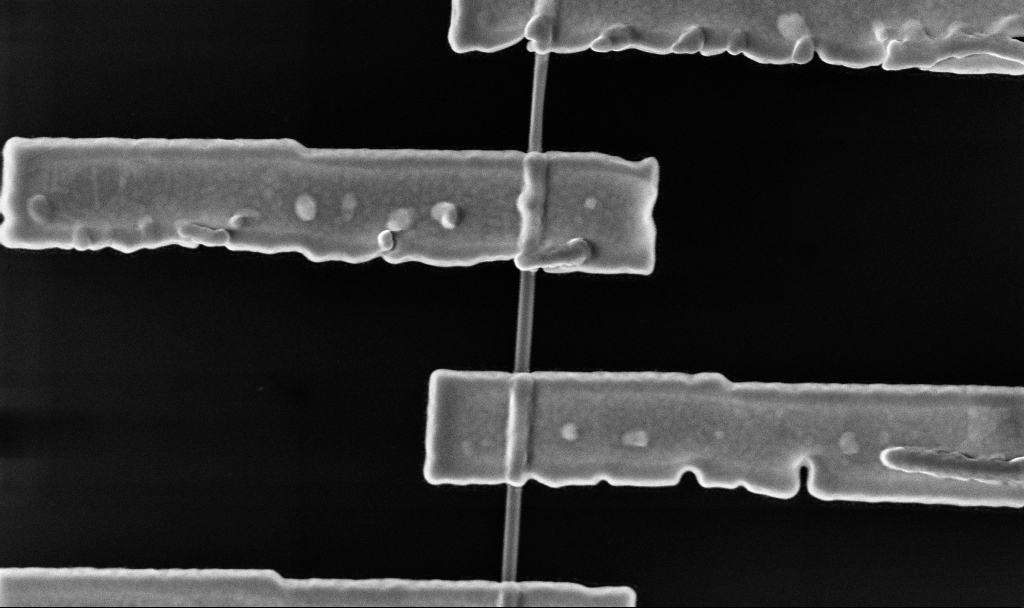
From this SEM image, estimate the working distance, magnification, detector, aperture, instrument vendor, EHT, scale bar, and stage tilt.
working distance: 6.7 mm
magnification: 80.23 K X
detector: InLens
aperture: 30 µm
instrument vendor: Zeiss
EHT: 10 kV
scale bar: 200 nm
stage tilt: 0°